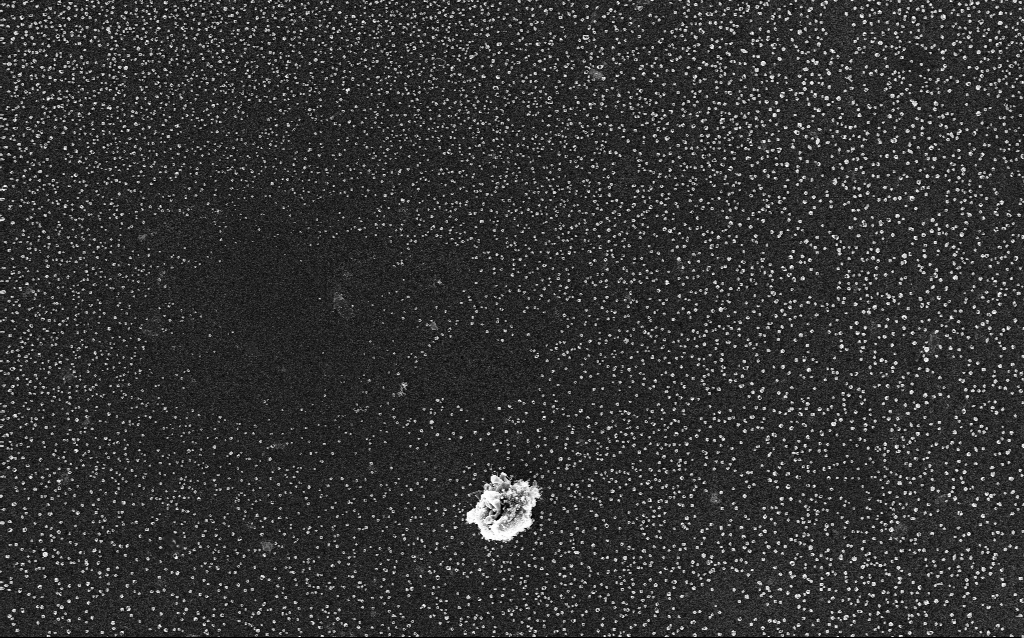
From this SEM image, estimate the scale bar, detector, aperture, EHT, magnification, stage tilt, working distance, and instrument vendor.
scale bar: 2000 nm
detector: InLens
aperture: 30 µm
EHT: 5 kV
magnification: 10 K X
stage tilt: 0°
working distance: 2.8 mm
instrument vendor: Zeiss